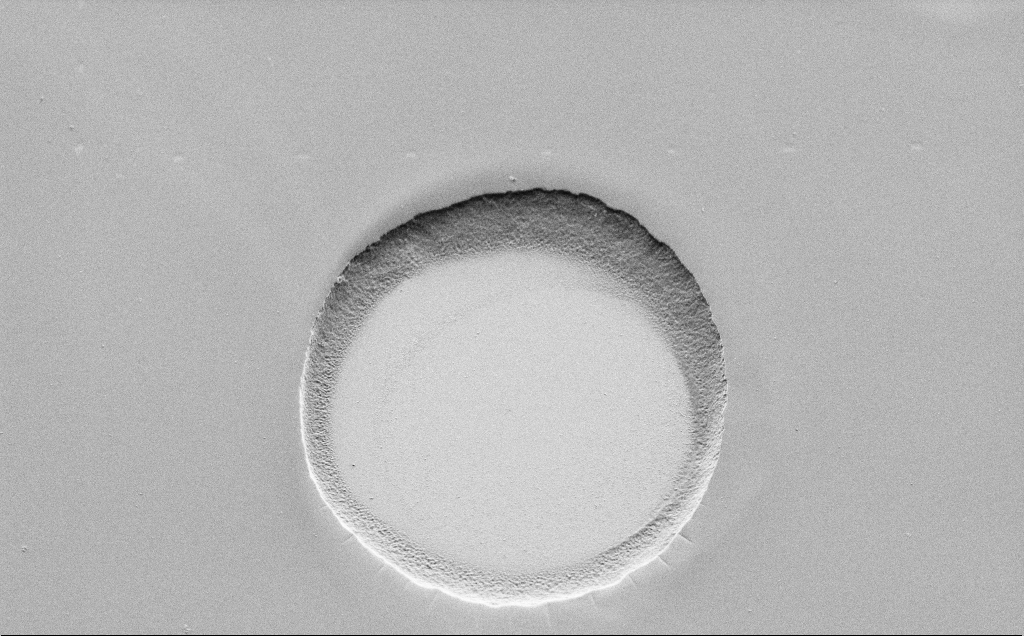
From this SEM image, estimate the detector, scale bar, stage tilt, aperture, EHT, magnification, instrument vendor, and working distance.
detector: SE2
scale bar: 10000 nm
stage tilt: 45°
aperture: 30 µm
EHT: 1.5 kV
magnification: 5.88 K X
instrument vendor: Zeiss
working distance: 6 mm